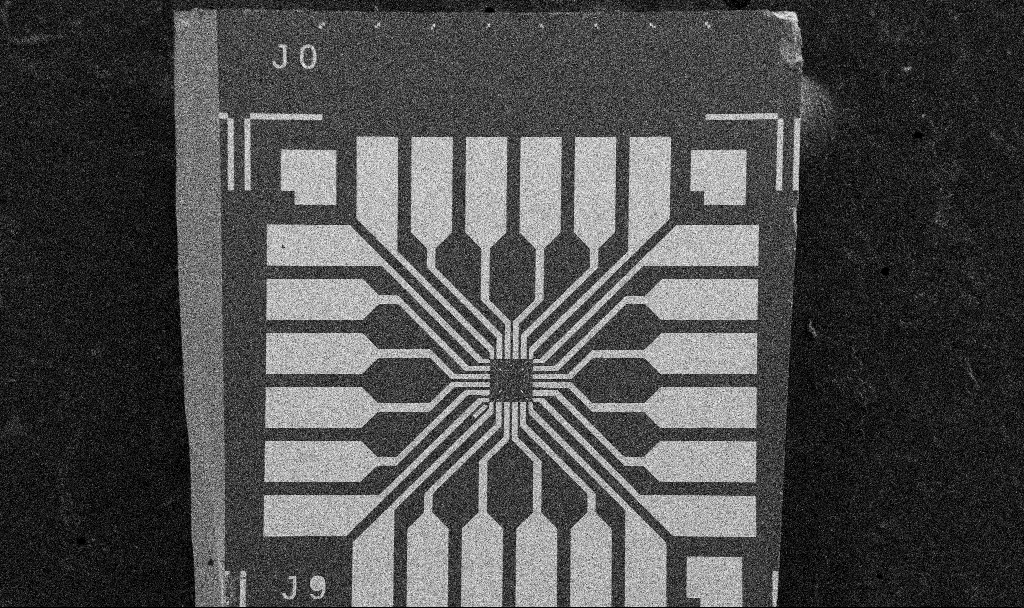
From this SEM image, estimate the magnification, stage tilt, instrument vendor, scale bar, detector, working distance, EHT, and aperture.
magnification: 0.1 K X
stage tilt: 0°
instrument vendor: Zeiss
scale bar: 200000 nm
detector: SE2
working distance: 8.7 mm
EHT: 5 kV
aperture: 30 µm